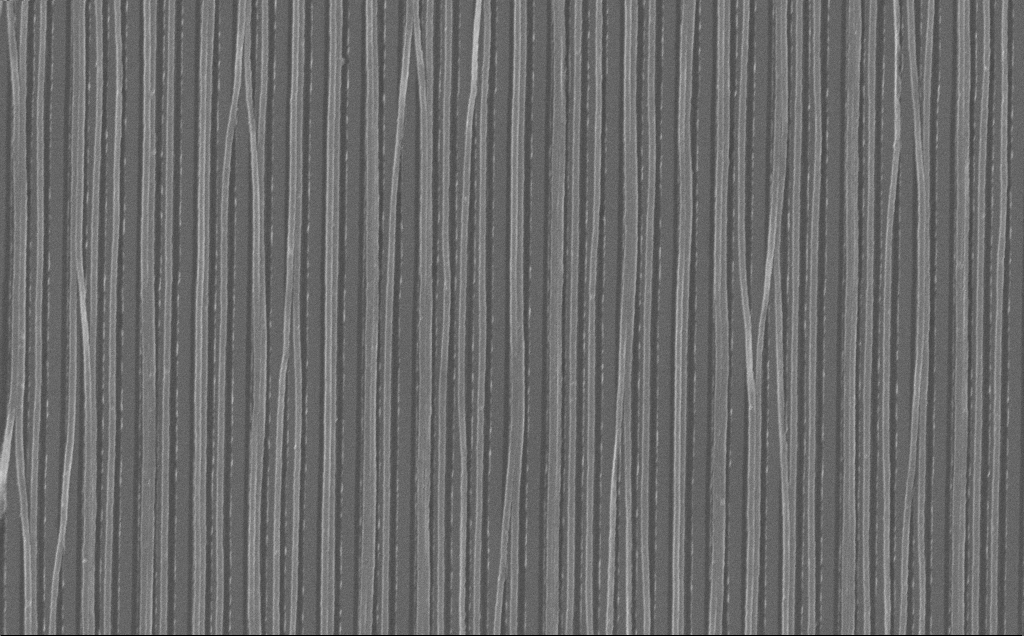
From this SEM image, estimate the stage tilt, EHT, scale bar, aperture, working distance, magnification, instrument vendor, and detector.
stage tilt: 0°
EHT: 10 kV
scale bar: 2000 nm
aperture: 30 µm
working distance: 7 mm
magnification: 27.21 K X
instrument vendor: Zeiss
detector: InLens